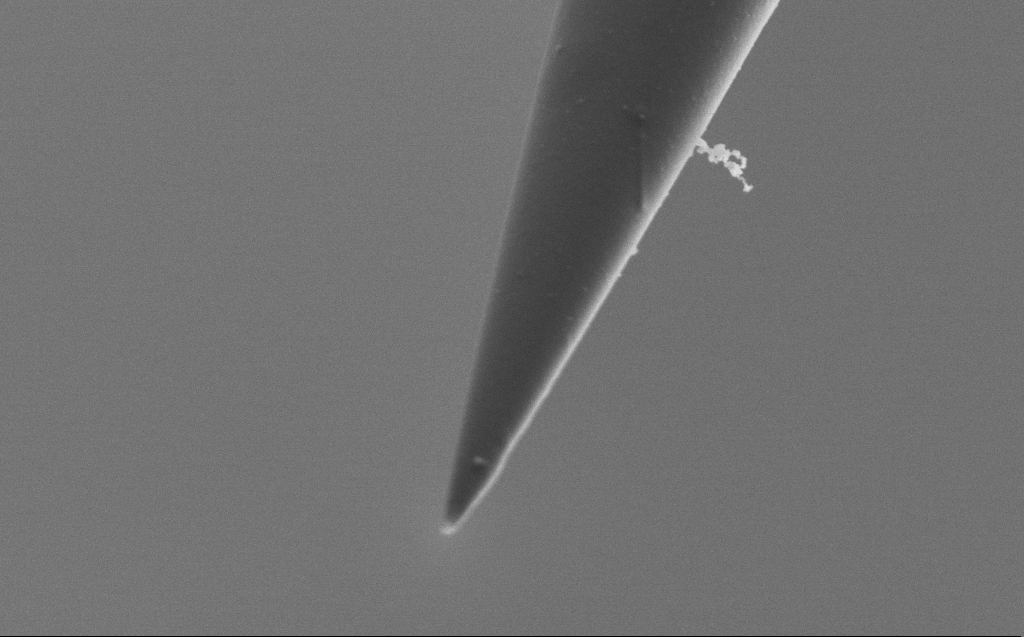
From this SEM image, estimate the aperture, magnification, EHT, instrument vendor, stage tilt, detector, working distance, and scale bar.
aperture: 30 µm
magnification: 50 K X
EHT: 2 kV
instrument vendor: Zeiss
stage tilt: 45°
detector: SE2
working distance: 4 mm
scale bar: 1000 nm